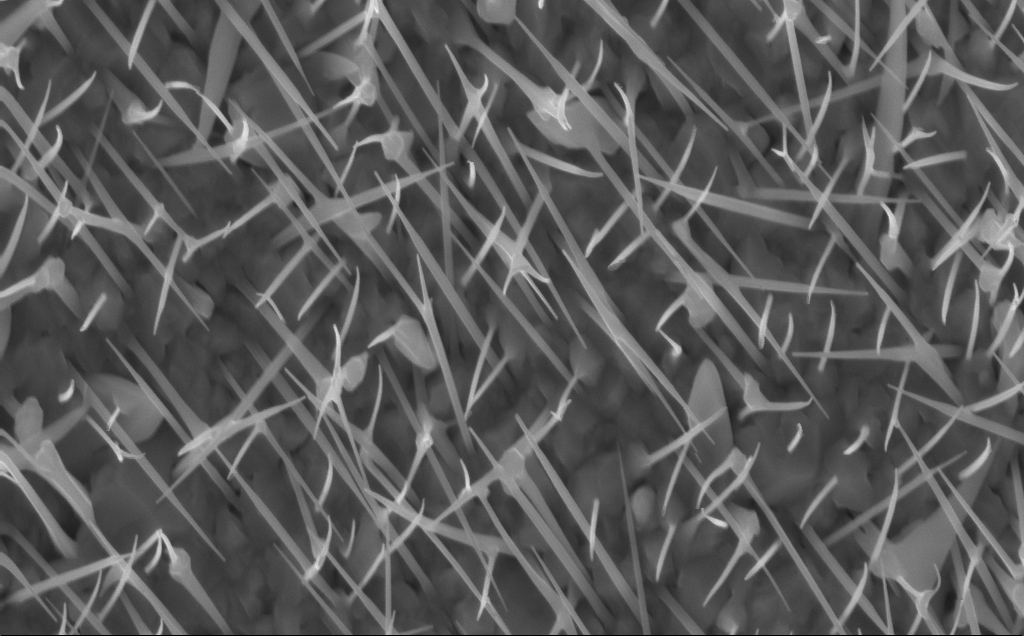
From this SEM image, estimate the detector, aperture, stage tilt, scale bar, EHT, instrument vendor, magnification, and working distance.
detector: InLens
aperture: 30 µm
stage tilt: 0°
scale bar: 1000 nm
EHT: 10 kV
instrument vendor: Zeiss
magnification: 40 K X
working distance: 5 mm